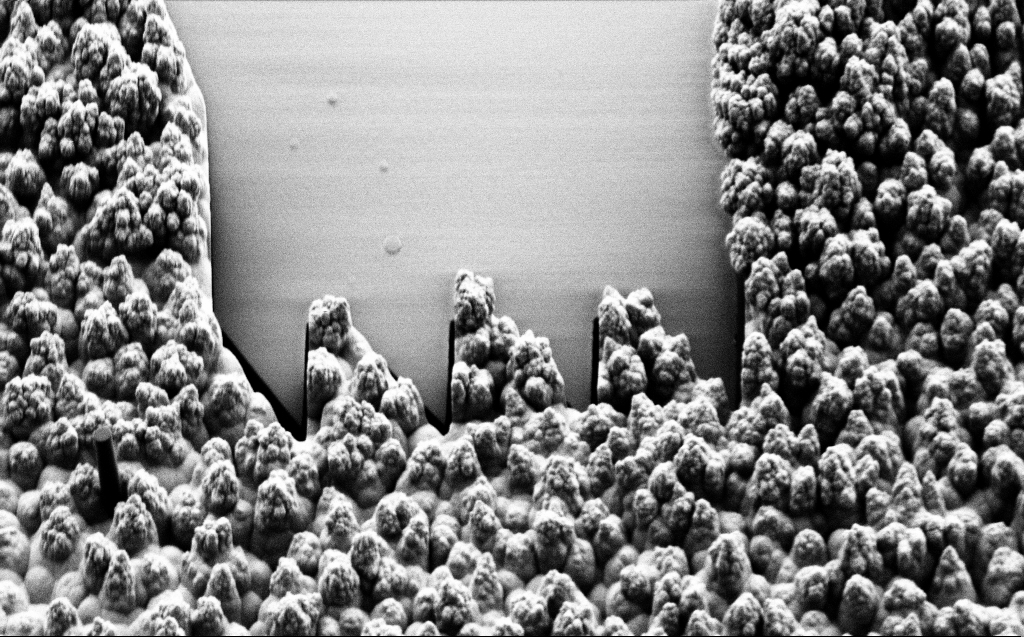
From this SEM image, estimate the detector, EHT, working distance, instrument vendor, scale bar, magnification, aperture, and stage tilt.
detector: SE2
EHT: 1 kV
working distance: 8 mm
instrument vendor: Zeiss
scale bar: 10000 nm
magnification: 4.64 K X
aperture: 30 µm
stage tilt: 45°